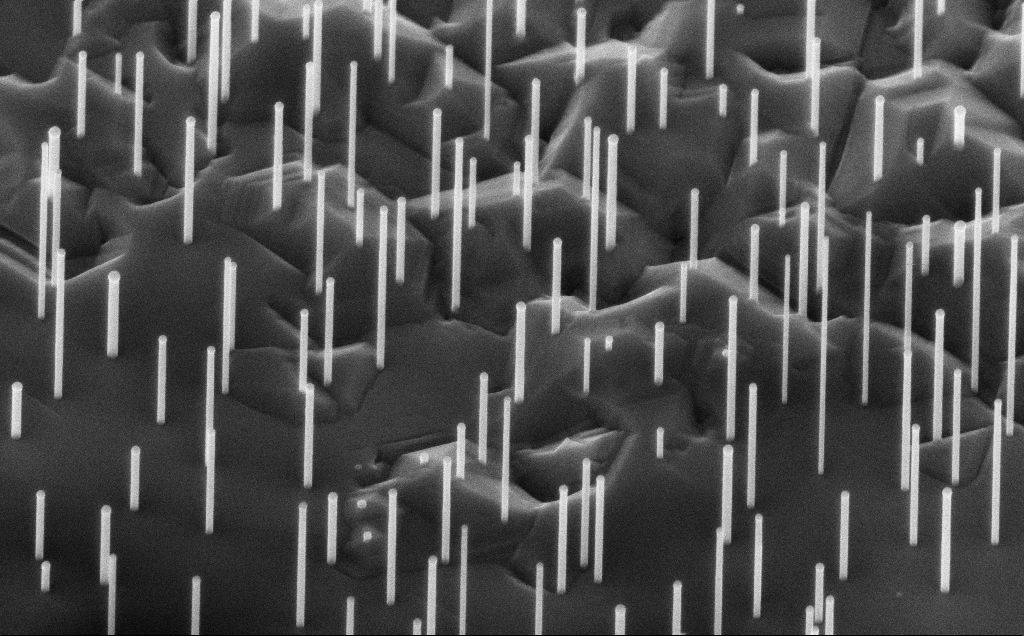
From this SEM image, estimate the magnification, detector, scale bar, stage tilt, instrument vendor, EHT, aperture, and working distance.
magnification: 50.46 K X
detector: InLens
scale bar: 1000 nm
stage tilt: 45°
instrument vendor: Zeiss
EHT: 10 kV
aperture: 30 µm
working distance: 6 mm